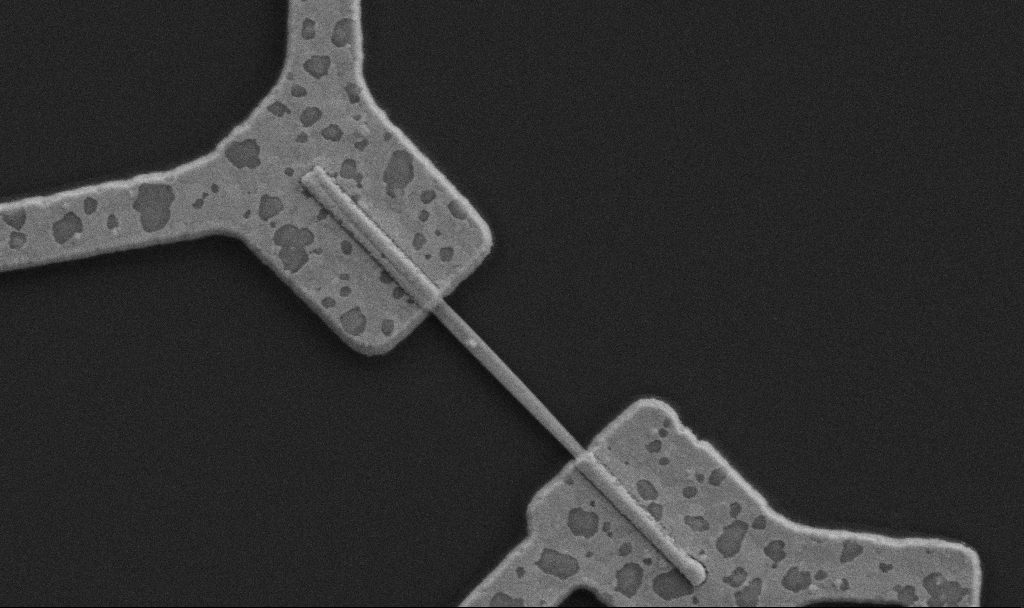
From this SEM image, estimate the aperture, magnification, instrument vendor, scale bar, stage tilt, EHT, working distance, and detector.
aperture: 30 µm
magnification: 30 K X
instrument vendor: Zeiss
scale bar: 2000 nm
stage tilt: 0°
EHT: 5 kV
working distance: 10.7 mm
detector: SE2